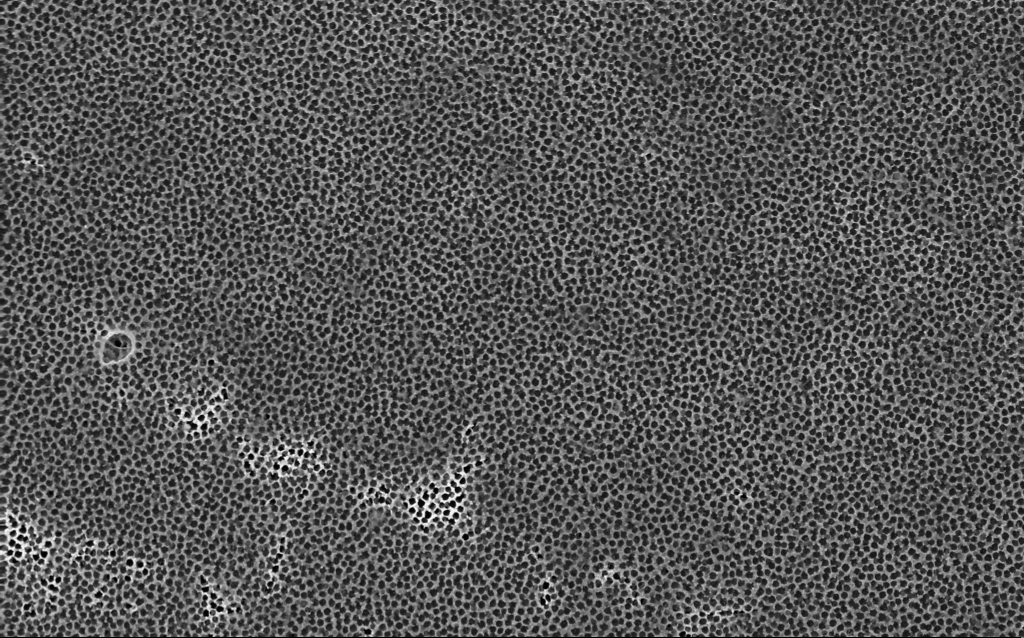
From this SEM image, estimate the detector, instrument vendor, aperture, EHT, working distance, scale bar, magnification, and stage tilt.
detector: InLens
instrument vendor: Zeiss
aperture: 30 µm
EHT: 5 kV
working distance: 3.9 mm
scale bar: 2000 nm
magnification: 10.71 K X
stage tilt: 0.1°